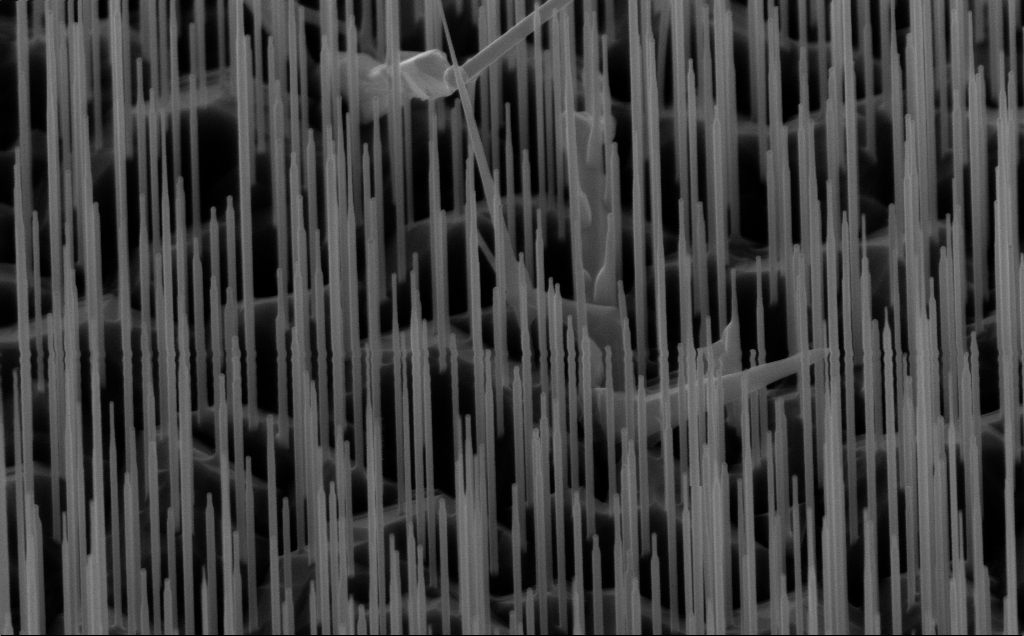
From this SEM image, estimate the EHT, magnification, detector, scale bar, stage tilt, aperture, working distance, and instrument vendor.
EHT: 10 kV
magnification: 40 K X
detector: InLens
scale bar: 1000 nm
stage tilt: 45°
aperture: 30 µm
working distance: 6 mm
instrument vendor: Zeiss